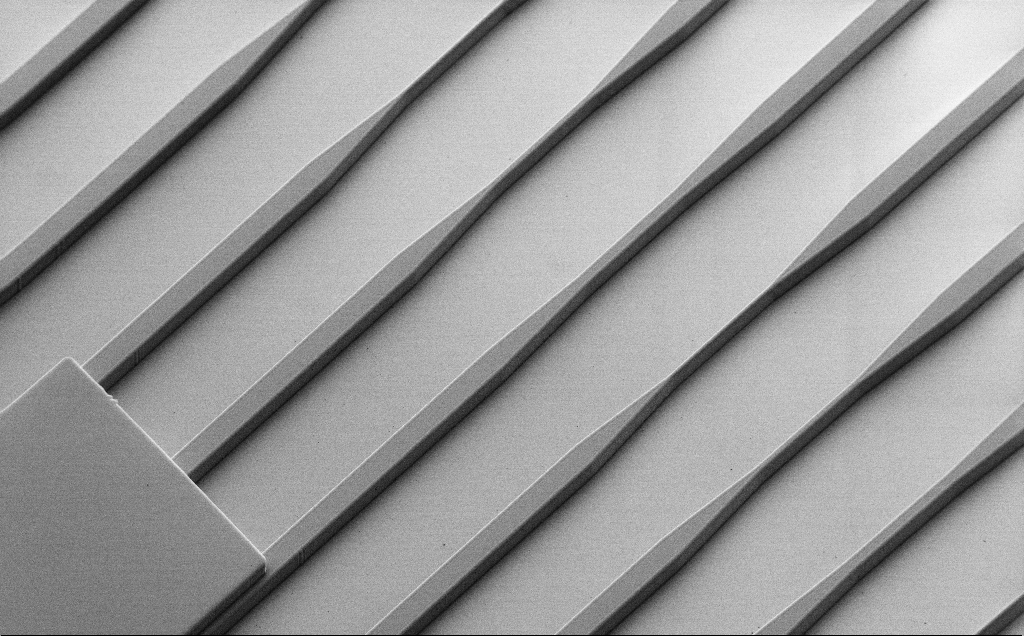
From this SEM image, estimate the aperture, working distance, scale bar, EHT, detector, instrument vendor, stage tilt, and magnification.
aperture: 30 µm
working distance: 9 mm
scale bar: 100000 nm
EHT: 1.3 kV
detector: SE2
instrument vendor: Zeiss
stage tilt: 45°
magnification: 0.455 K X